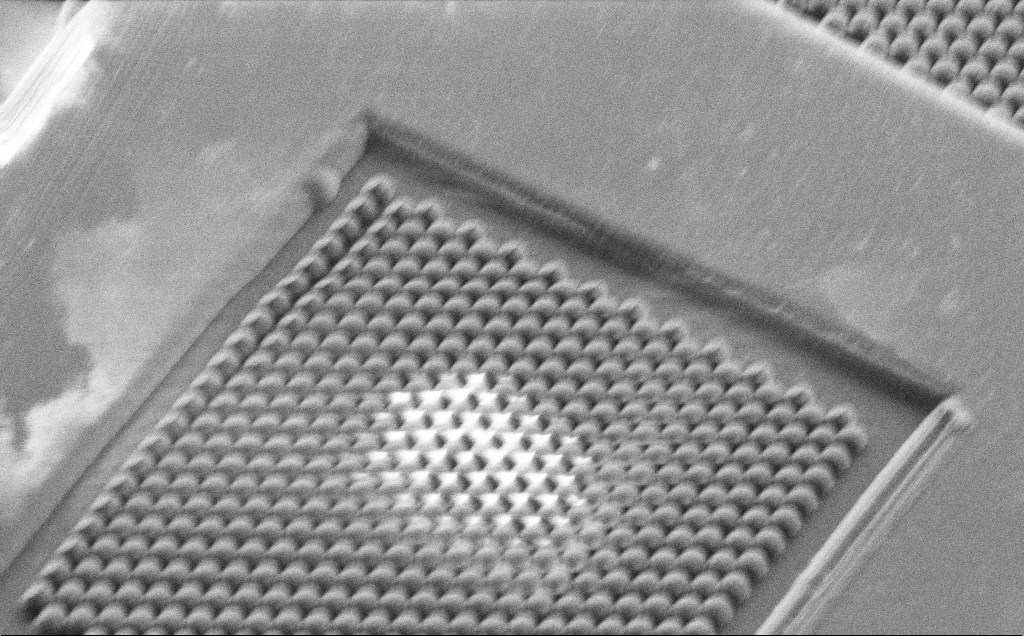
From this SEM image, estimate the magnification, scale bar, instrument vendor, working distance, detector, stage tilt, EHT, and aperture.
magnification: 2.72 K X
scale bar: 20000 nm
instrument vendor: Zeiss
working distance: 4 mm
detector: SE2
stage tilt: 32.7°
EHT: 2.4 kV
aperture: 30 µm